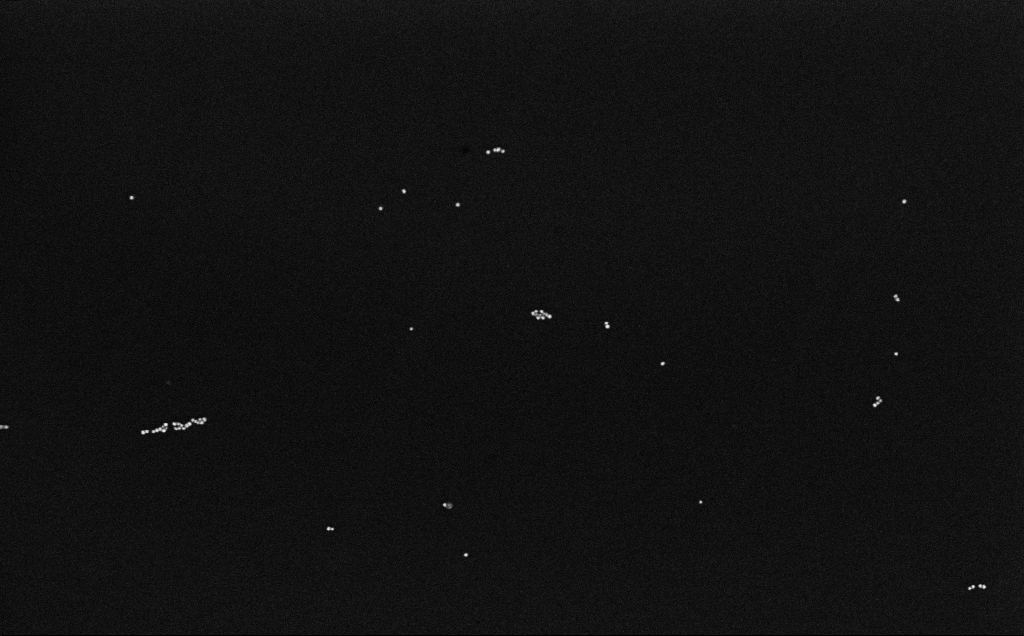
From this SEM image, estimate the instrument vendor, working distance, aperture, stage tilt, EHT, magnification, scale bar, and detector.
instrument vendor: Zeiss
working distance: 6.6 mm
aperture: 30 µm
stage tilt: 0°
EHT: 10 kV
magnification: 100 K X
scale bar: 200 nm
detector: InLens